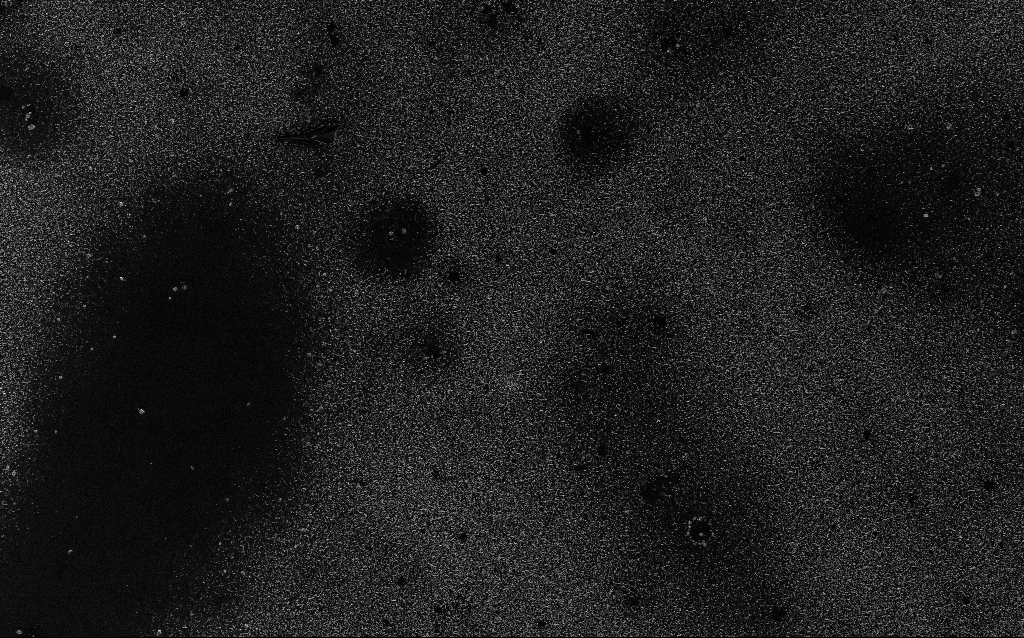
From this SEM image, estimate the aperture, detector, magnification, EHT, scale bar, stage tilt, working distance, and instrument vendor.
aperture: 30 µm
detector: InLens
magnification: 2 K X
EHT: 5 kV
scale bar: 10000 nm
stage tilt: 0°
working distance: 2.2 mm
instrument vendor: Zeiss